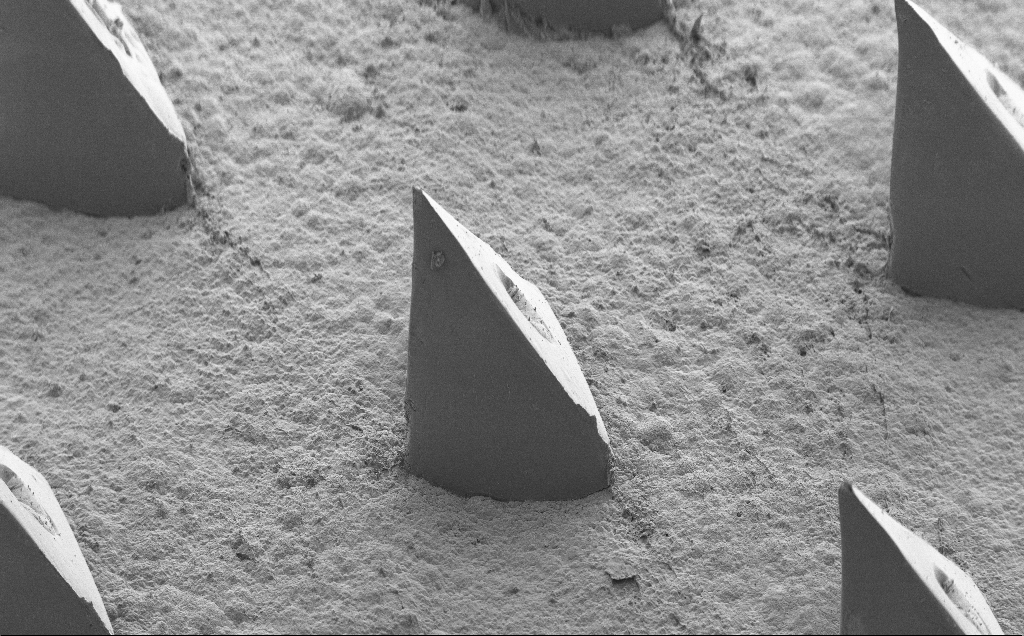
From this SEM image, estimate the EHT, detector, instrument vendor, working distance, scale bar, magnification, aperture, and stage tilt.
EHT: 5 kV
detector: SE2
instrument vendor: Zeiss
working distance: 9 mm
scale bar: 200000 nm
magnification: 0.132 K X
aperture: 30 µm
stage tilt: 40°